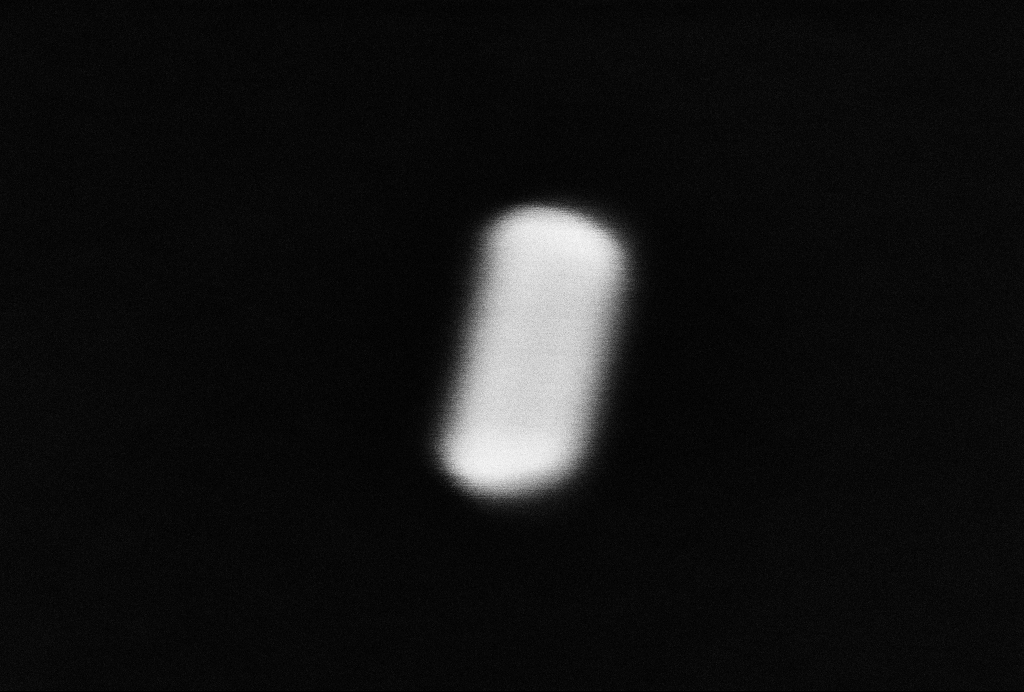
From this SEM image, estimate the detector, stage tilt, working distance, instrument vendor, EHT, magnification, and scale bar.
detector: InLens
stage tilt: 0°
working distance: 3.2 mm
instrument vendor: Zeiss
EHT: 10 kV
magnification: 1667.94 K X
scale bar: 20 nm